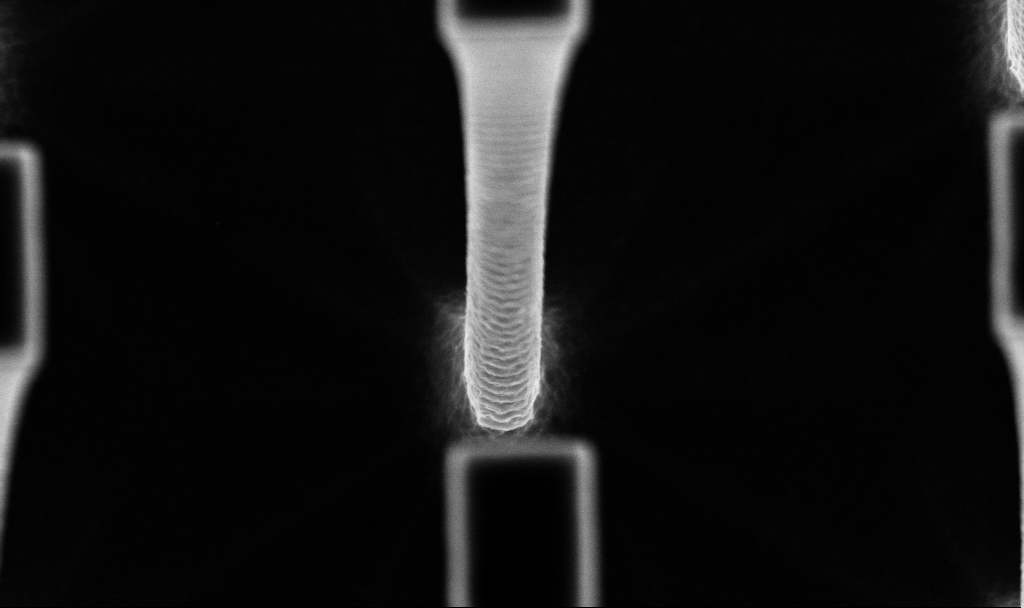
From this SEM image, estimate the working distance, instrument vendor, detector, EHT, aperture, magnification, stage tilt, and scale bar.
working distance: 4.8 mm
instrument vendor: Zeiss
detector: InLens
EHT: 5 kV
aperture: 30 µm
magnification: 36.71 K X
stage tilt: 12°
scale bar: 1000 nm